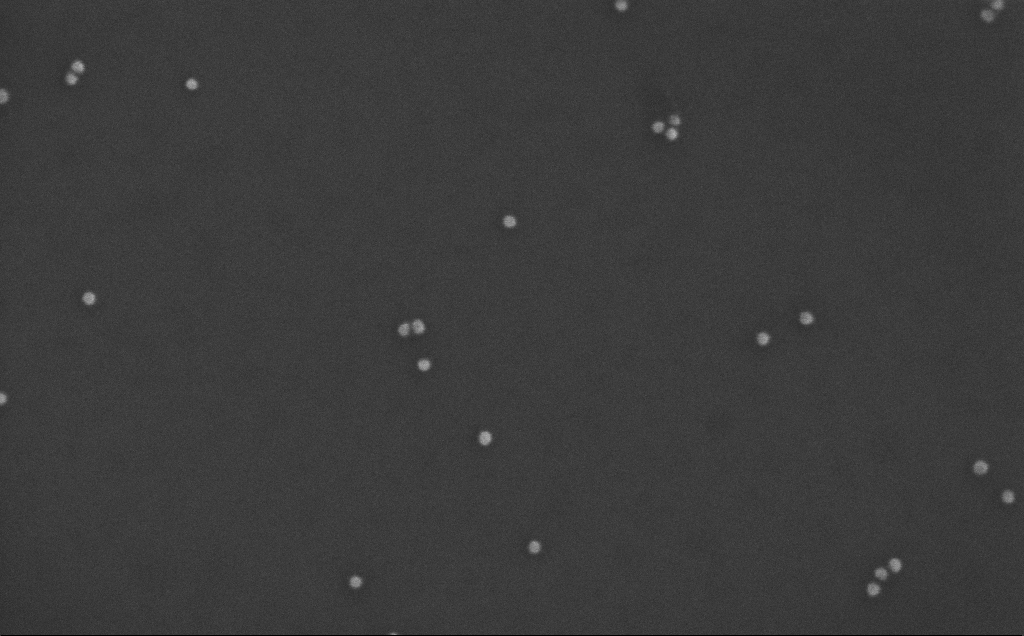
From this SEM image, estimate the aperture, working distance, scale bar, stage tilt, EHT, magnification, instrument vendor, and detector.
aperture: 30 µm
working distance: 3 mm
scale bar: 200 nm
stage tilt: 0°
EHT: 10 kV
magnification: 268.52 K X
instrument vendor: Zeiss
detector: InLens